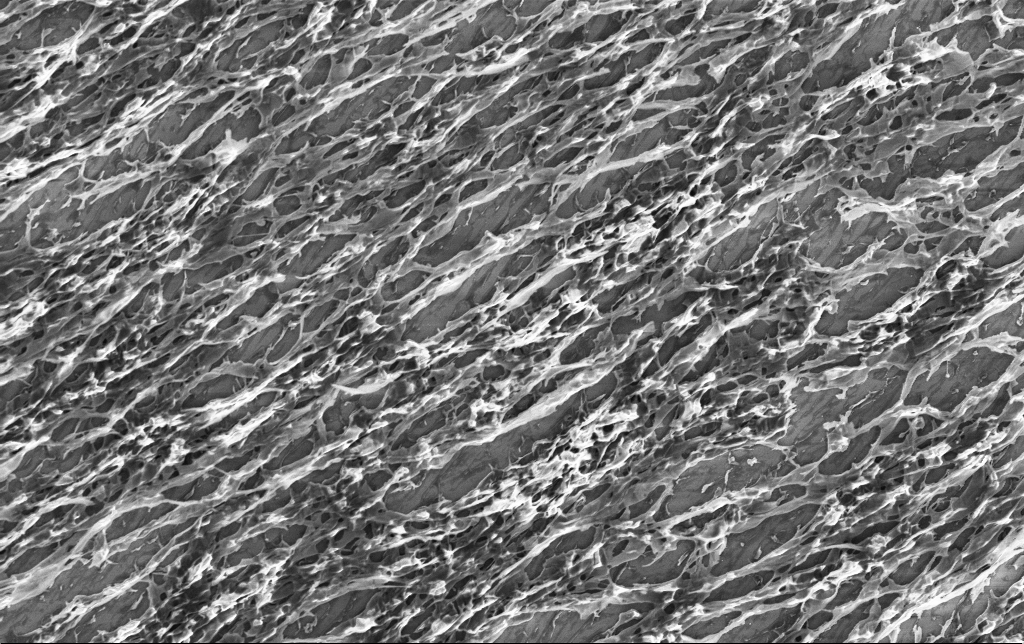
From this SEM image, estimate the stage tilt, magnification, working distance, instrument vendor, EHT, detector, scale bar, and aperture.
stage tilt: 0°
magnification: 4.59 K X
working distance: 3.2 mm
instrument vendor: Zeiss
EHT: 3 kV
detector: InLens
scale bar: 10000 nm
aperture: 30 µm